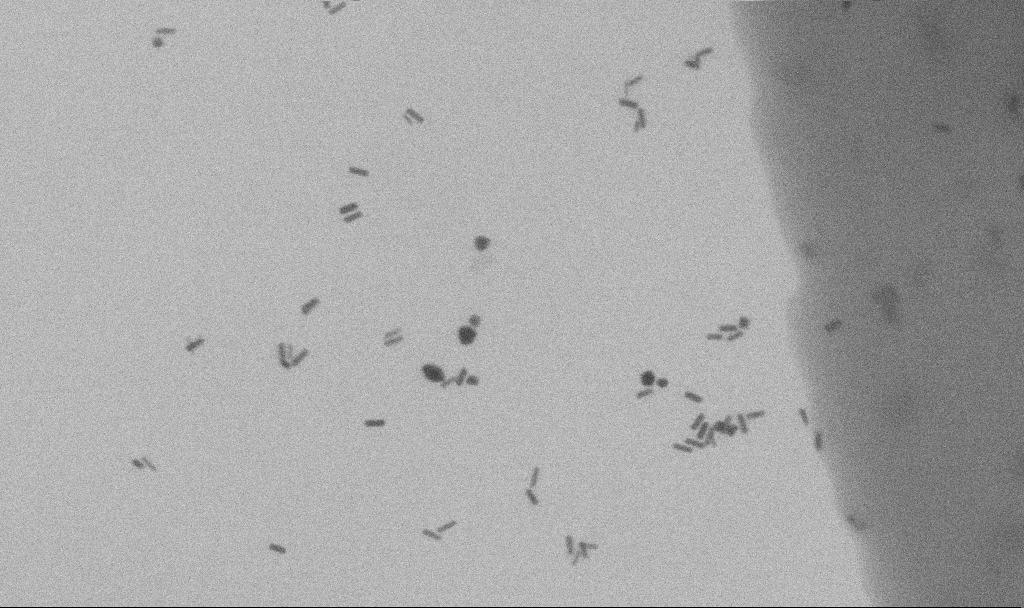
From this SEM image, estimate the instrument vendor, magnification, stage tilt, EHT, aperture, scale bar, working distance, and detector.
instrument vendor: Zeiss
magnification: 100 K X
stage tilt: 0°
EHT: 10 kV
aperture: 30 µm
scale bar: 200 nm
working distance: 11.3 mm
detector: SE2